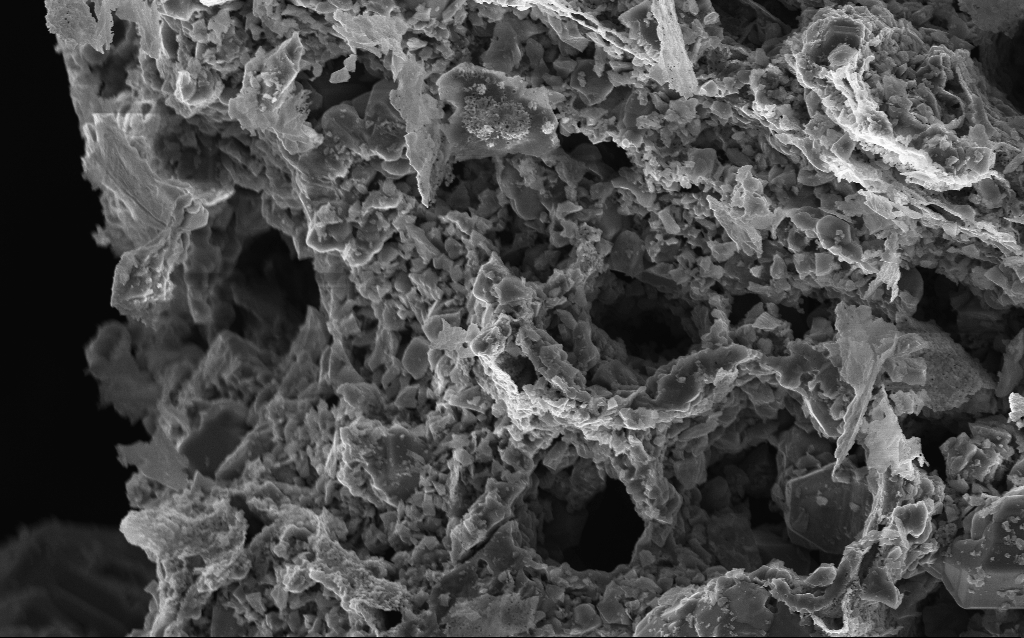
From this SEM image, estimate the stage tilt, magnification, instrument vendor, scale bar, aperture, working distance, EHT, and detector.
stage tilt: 0°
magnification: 5 K X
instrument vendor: Zeiss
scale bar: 10000 nm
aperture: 30 µm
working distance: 3 mm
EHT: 10 kV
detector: InLens